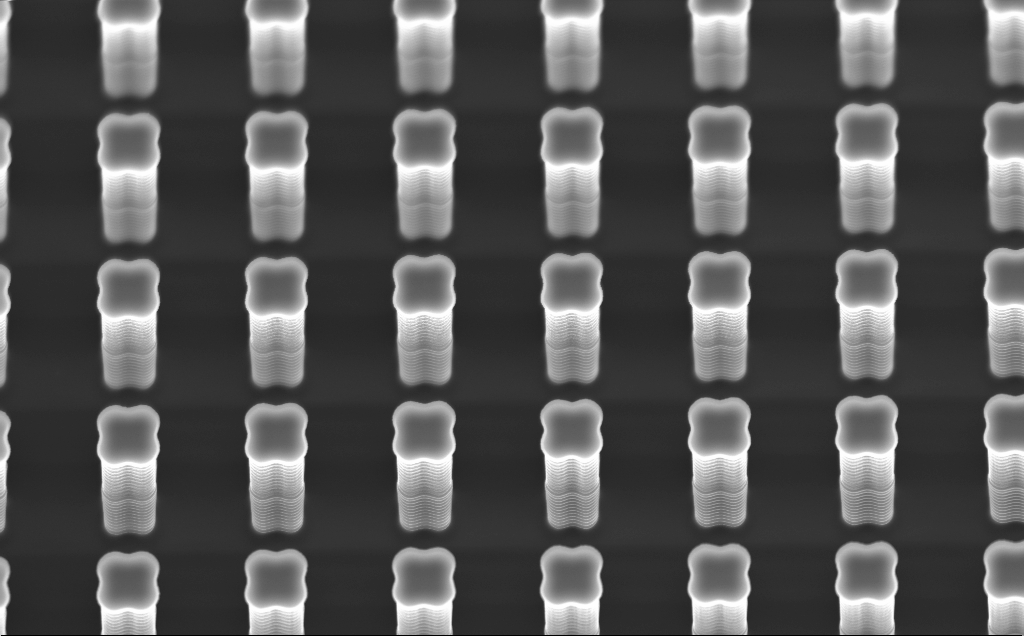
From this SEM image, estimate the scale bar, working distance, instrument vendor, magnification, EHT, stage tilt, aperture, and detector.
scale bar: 10000 nm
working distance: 9 mm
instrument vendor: Zeiss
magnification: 5.43 K X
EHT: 10 kV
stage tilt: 30.3°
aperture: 120 µm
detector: InLens